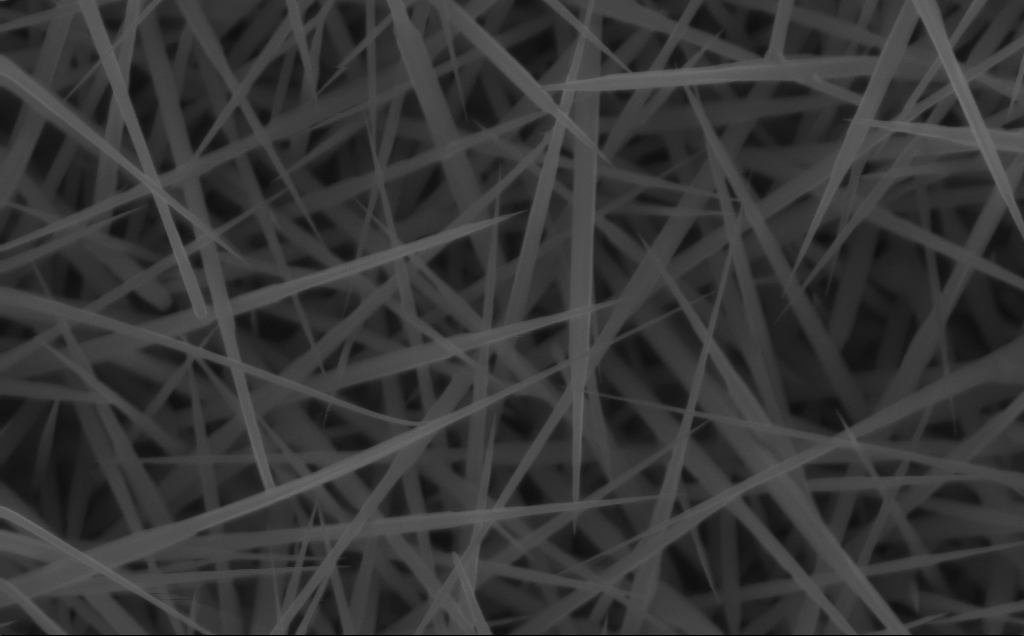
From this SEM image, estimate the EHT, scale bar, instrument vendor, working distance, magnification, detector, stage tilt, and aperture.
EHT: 10 kV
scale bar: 200 nm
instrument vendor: Zeiss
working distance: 4 mm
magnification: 80 K X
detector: InLens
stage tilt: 0°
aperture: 30 µm